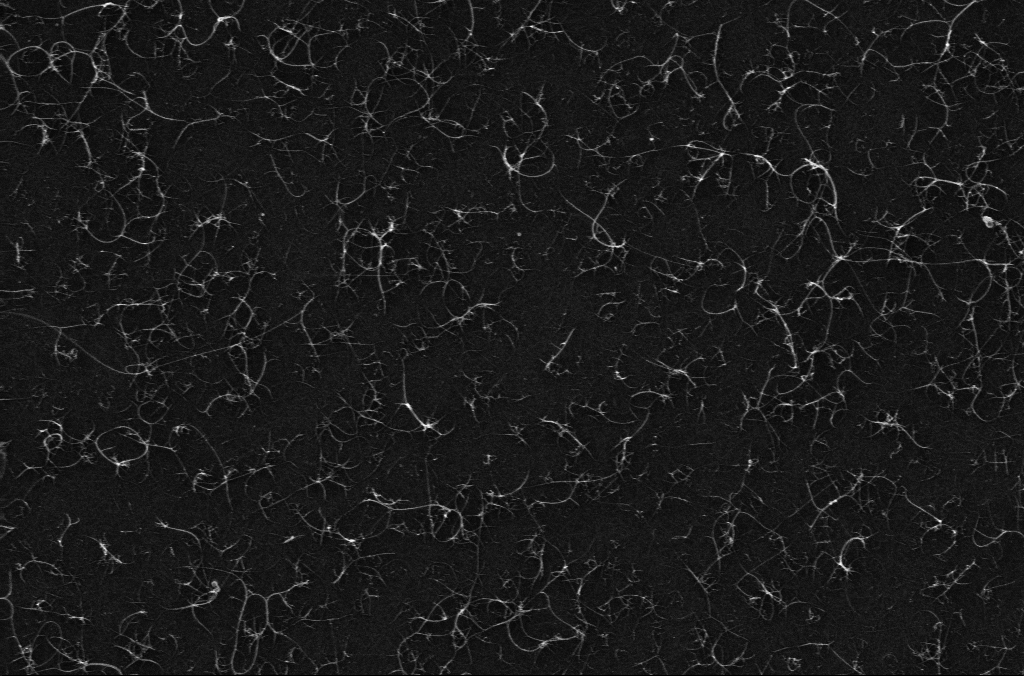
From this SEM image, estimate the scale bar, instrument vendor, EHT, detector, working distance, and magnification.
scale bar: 200 nm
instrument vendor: Zeiss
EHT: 10 kV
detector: InLens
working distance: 3.2 mm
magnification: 67.14 K X